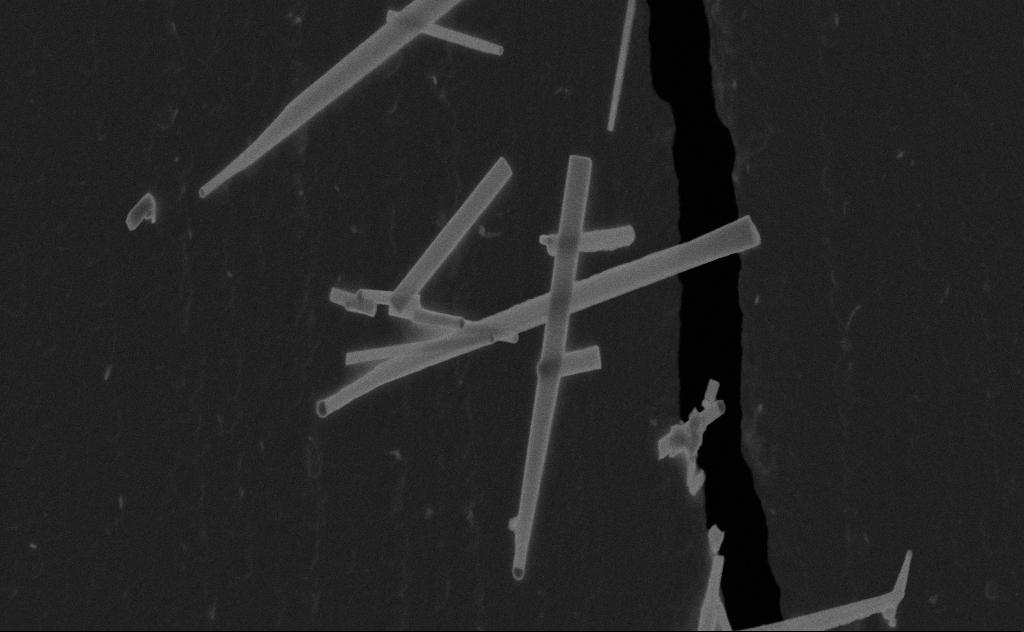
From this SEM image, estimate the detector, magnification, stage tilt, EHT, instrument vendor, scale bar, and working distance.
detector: SE2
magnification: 70.07 K X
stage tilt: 0°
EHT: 20 kV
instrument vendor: Zeiss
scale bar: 1000 nm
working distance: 9 mm